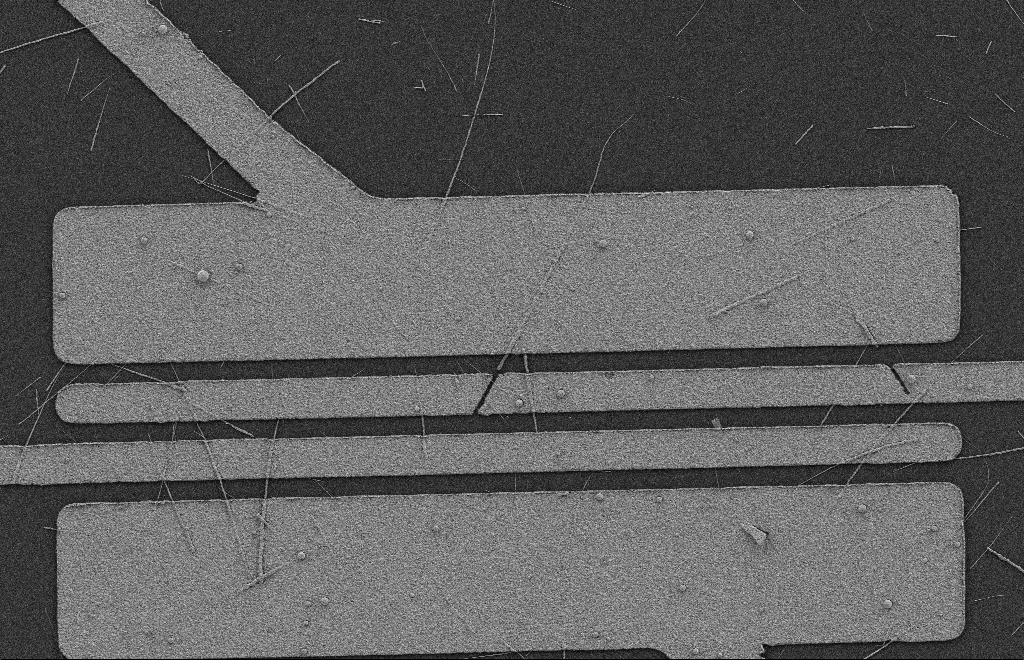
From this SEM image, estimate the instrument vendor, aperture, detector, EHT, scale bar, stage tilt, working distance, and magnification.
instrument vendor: Zeiss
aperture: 20 µm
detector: SE2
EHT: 2 kV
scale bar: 2000 nm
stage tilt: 0°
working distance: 8 mm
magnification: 5.44 K X